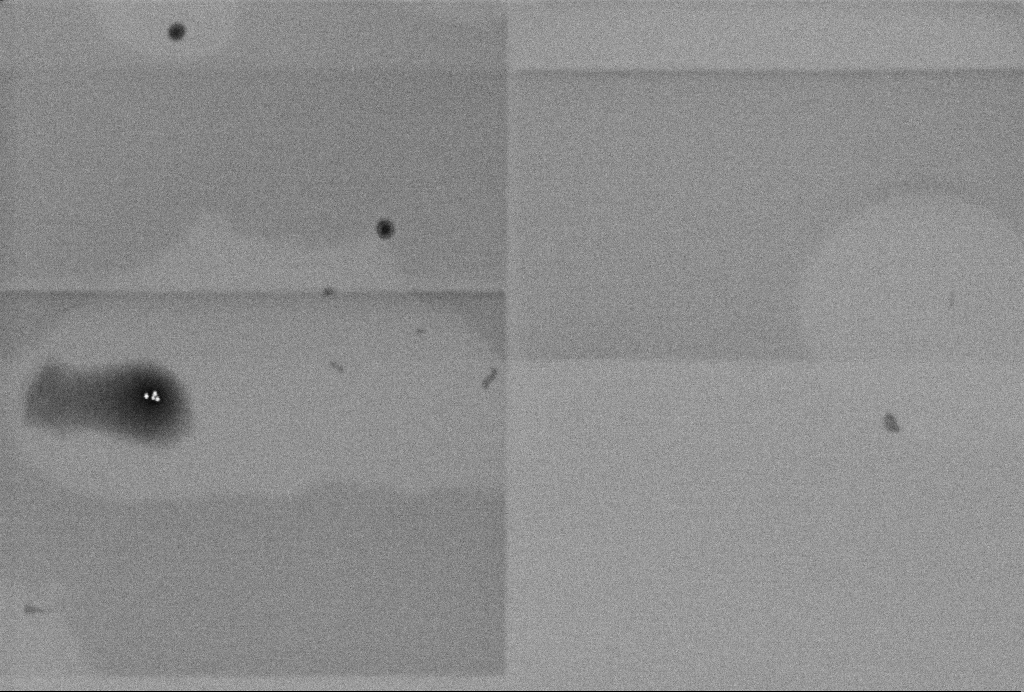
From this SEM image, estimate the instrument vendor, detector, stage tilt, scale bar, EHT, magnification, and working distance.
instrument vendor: Zeiss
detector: InLens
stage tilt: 0°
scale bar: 200 nm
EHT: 1 kV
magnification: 65 K X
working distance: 3.3 mm